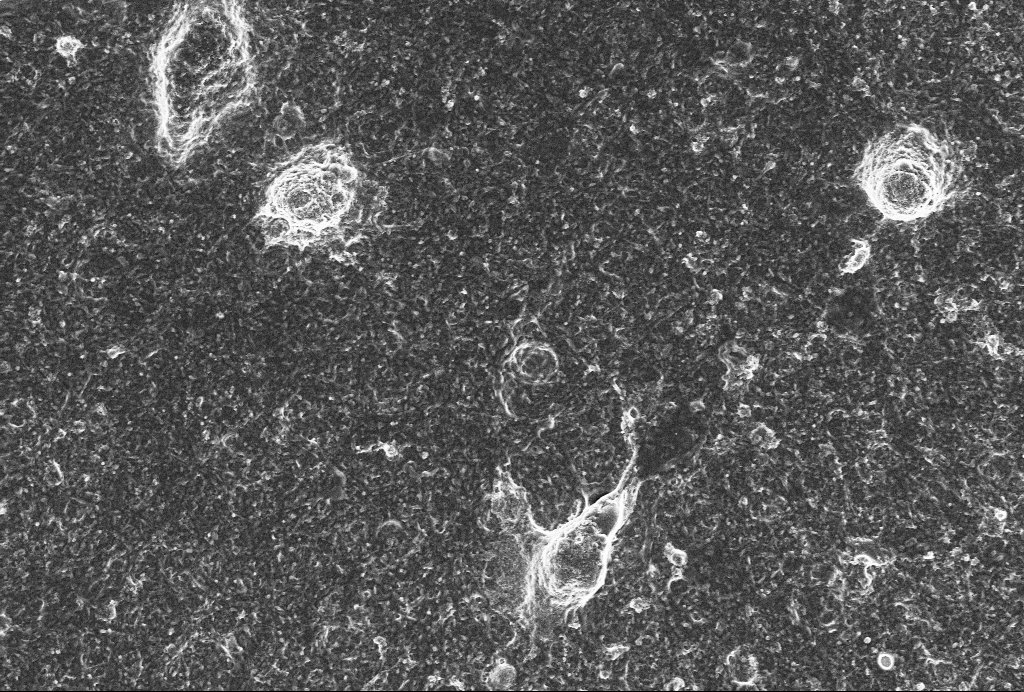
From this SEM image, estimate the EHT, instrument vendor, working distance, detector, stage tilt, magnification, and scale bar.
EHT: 4 kV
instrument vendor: Zeiss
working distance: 6 mm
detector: InLens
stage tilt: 0°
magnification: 4 K X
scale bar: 10000 nm